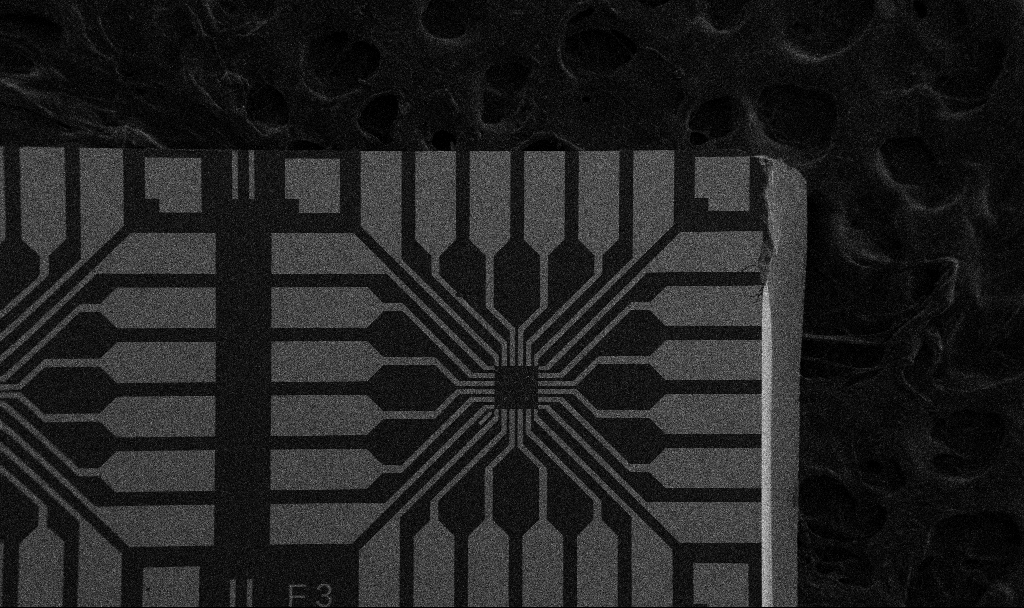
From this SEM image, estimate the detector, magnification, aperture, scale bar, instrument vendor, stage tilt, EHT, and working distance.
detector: SE2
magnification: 0.1 K X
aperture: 30 µm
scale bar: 200000 nm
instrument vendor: Zeiss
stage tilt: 0°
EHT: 5 kV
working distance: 10.7 mm